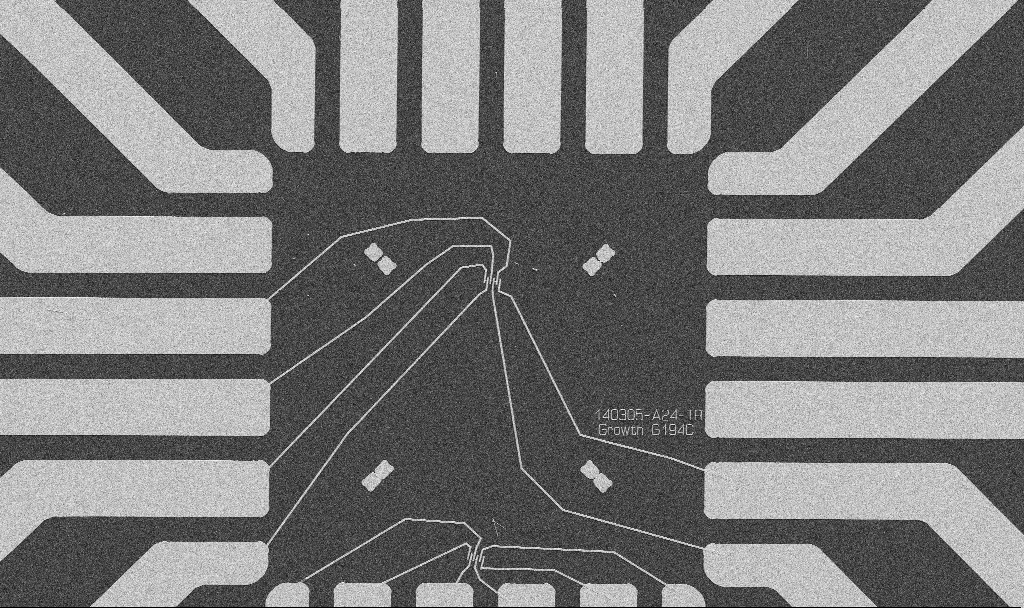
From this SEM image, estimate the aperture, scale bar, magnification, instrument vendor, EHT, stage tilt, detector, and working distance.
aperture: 30 µm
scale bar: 20000 nm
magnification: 1 K X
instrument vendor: Zeiss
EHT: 5 kV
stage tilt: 0°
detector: SE2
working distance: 10.7 mm